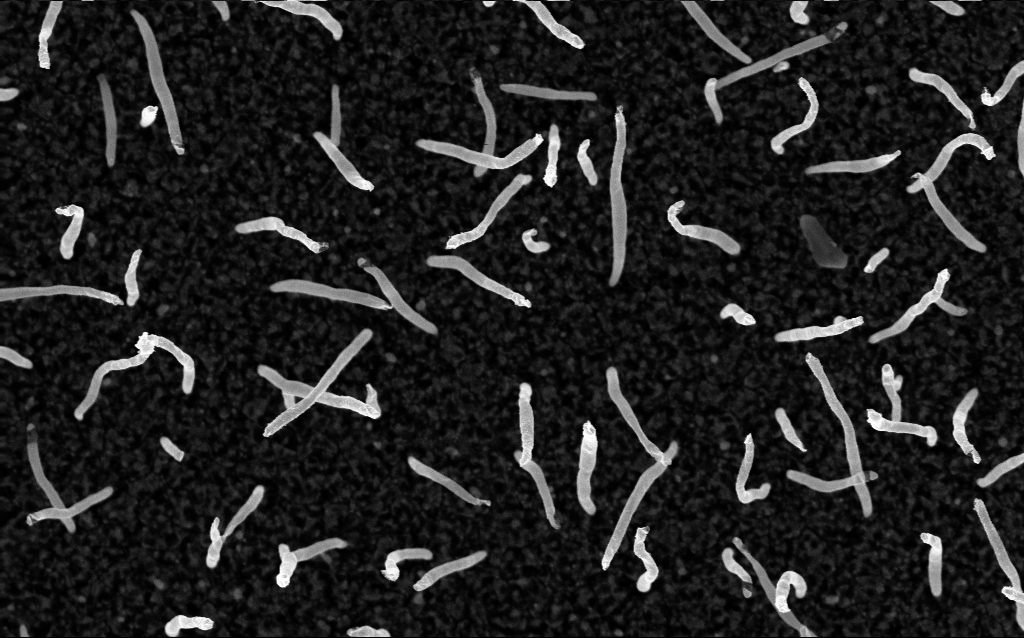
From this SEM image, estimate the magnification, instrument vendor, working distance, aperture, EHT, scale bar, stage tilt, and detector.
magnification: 50 K X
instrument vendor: Zeiss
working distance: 2 mm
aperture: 30 µm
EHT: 5 kV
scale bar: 1000 nm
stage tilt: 0°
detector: InLens